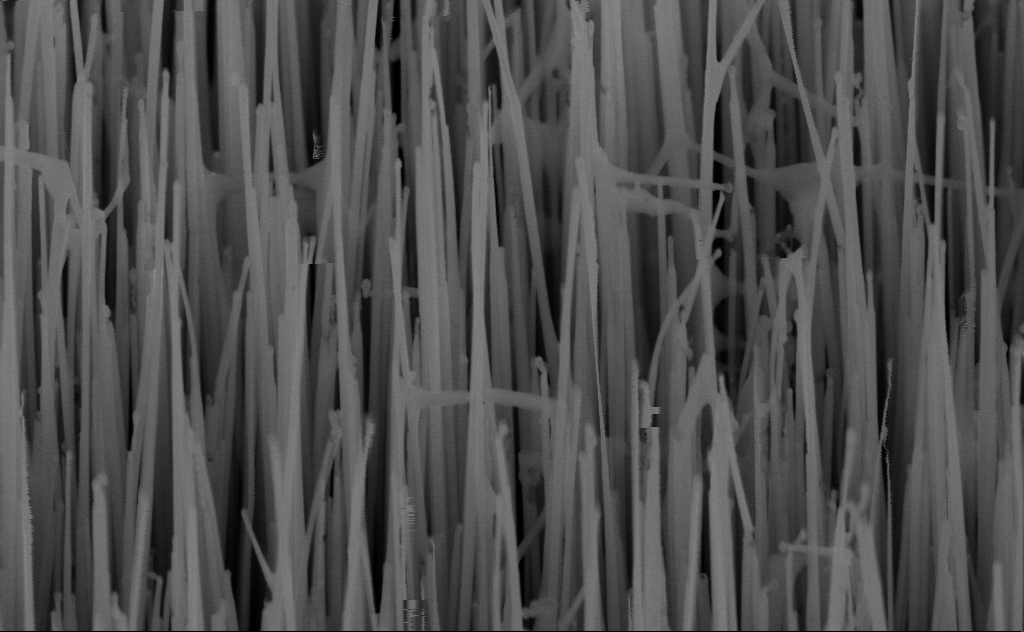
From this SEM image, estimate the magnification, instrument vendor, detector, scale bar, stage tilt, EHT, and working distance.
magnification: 80 K X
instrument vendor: Zeiss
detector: InLens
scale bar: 200 nm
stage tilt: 45°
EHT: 10 kV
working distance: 6 mm